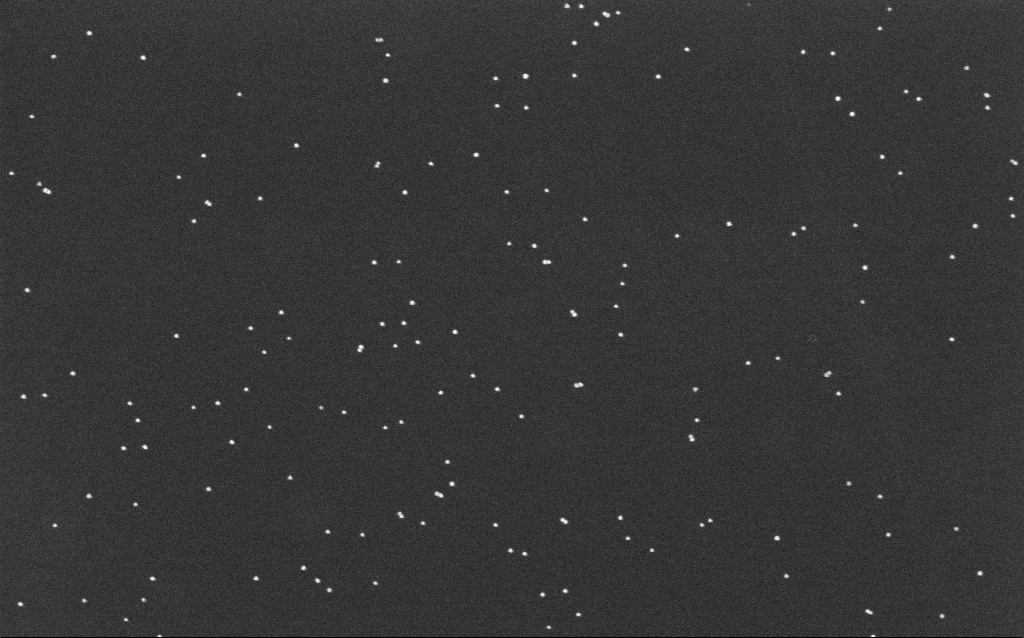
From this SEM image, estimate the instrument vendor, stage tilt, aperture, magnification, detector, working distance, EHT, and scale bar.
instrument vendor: Zeiss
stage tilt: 0°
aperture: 30 µm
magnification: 100 K X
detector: InLens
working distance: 6.4 mm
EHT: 10 kV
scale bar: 200 nm